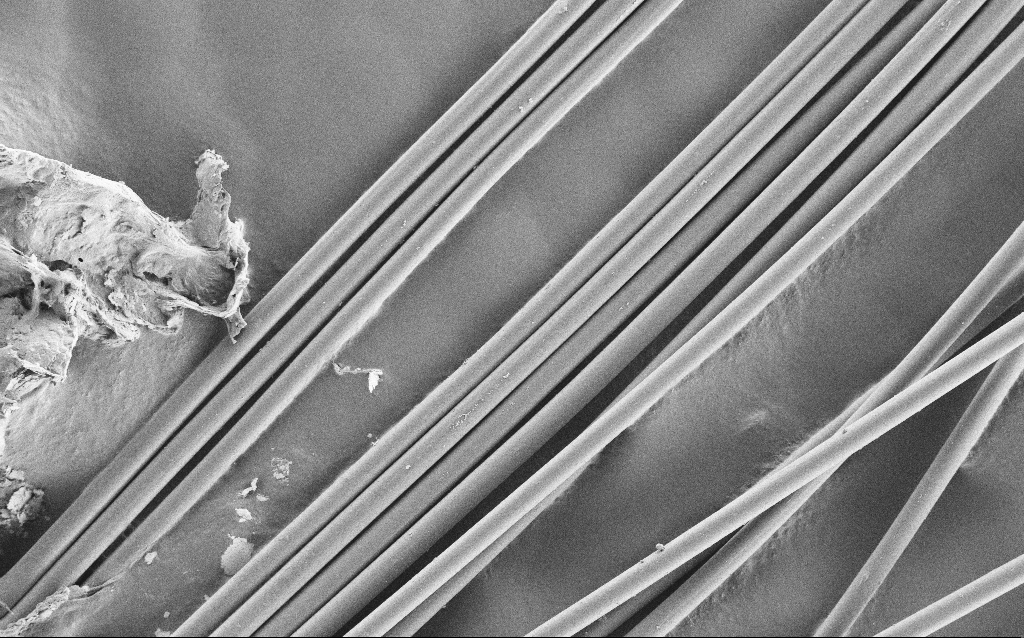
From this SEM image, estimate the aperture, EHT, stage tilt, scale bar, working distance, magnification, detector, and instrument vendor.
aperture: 30 µm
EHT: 1 kV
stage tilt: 0°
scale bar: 20000 nm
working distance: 5 mm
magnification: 0.73 K X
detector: SE2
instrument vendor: Zeiss